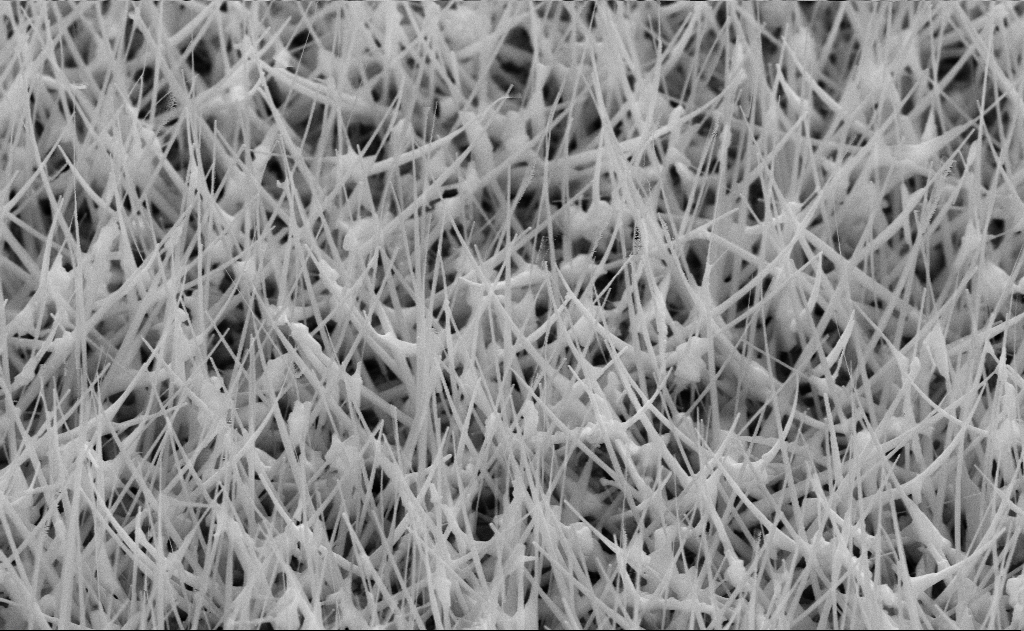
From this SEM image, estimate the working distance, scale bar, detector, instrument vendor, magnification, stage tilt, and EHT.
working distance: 11 mm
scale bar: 1000 nm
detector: SE2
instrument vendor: Zeiss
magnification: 40 K X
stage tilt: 45°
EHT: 10 kV